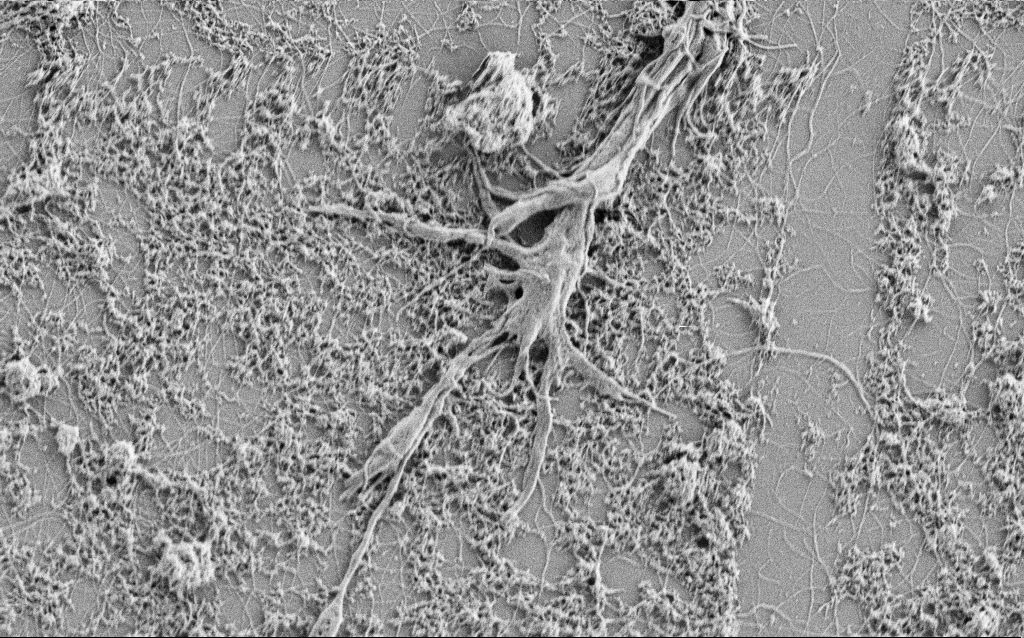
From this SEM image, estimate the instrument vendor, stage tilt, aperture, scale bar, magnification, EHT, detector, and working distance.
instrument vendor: Zeiss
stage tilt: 0°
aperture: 30 µm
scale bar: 2000 nm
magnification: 10 K X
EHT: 1 kV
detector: SE2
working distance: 4 mm